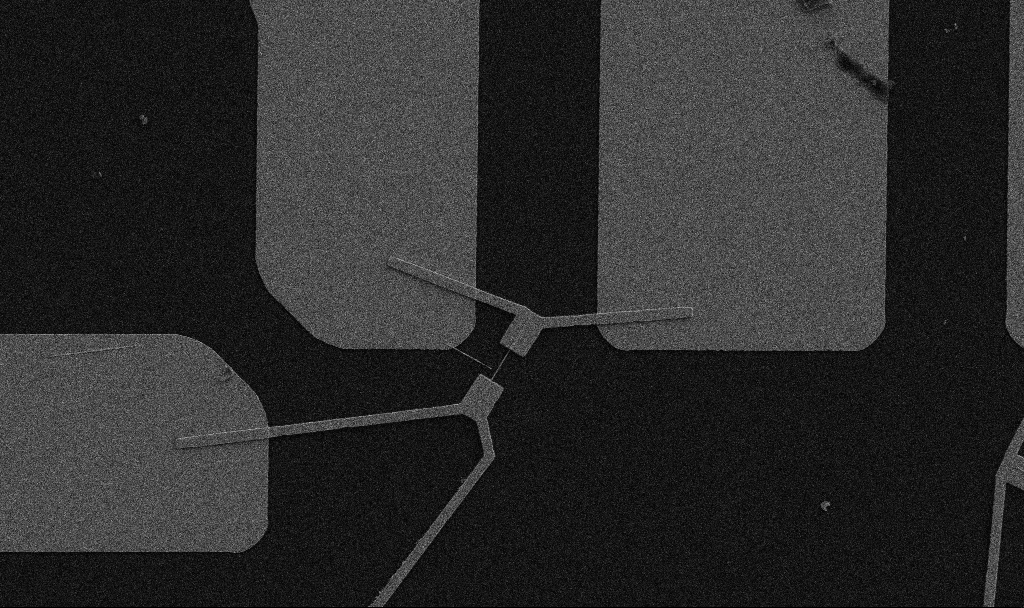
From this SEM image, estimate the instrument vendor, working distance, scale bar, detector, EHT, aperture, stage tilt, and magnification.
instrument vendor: Zeiss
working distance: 10.7 mm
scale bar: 10000 nm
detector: SE2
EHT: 5 kV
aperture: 30 µm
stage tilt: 0°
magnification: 5 K X